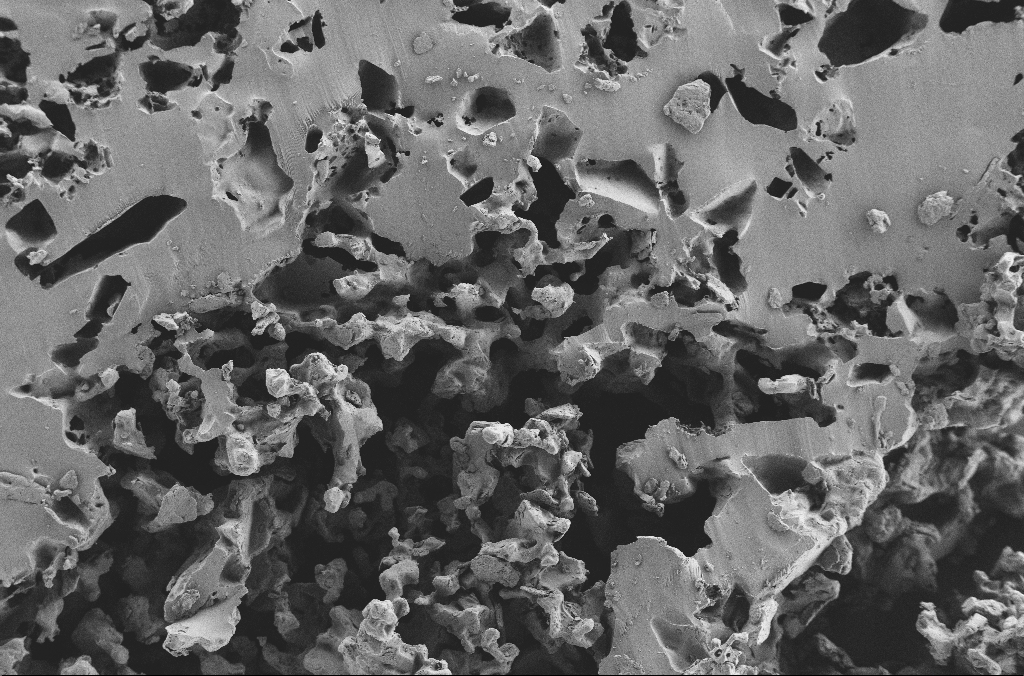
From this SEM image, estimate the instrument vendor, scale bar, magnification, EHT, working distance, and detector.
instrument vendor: Zeiss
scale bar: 100000 nm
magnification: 0.25 K X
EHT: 2 kV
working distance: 3.1 mm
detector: SE2